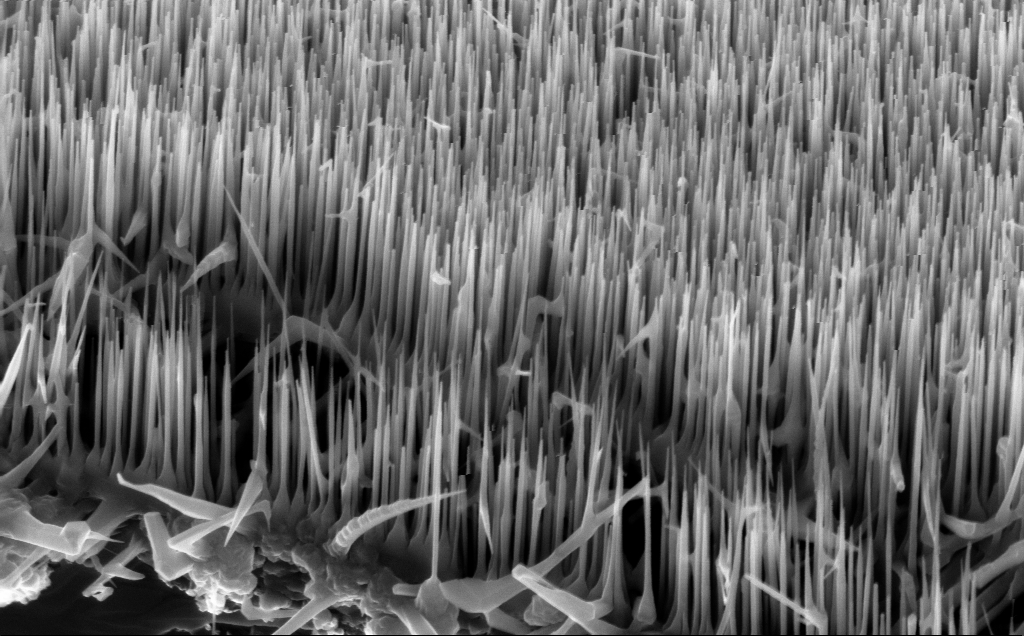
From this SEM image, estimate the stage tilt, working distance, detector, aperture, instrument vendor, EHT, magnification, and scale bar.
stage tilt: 45°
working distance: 5 mm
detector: InLens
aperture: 30 µm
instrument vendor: Zeiss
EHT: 10 kV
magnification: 49.59 K X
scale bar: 1000 nm